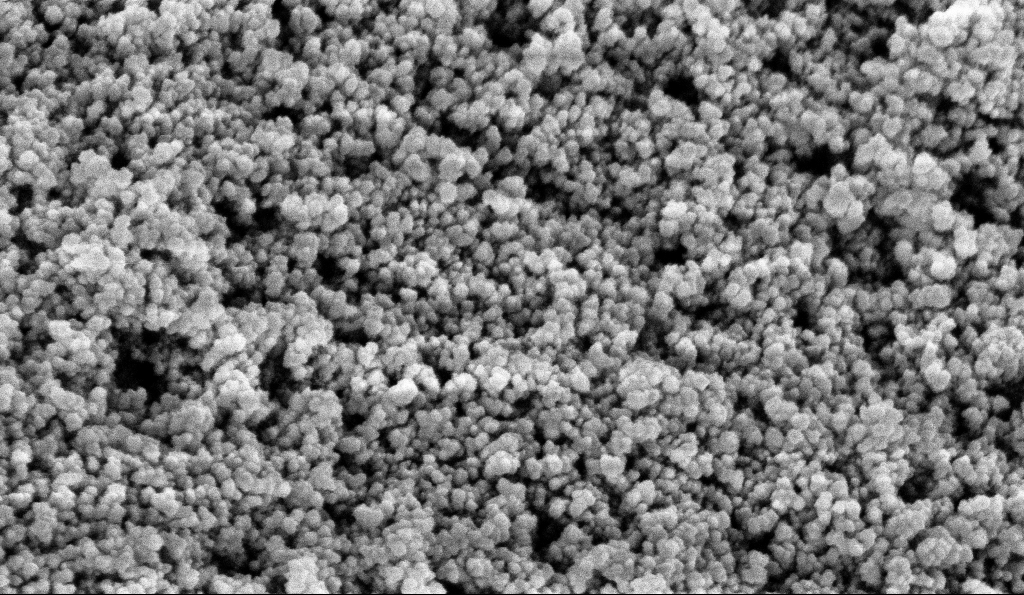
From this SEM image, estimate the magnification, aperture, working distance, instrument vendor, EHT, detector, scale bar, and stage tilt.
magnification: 135 K X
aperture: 30 µm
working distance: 5.9 mm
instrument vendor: Zeiss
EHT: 5 kV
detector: InLens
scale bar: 100 nm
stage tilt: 0°